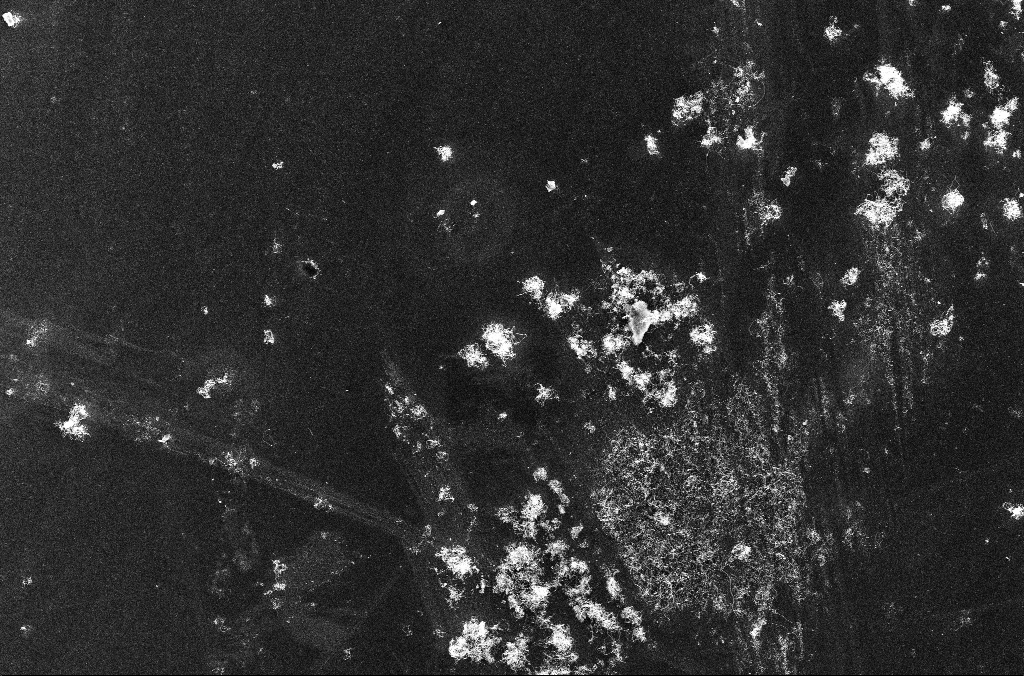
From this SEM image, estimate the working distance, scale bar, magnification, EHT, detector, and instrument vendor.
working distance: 3.3 mm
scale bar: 10000 nm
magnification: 1.5 K X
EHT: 10 kV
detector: InLens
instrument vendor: Zeiss